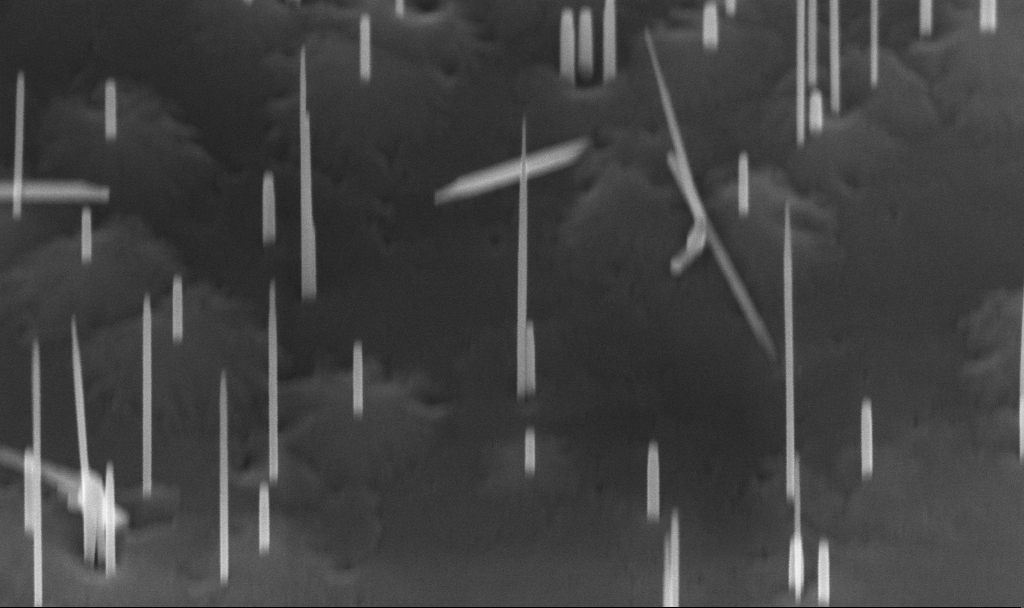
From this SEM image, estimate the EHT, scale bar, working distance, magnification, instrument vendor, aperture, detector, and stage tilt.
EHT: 10 kV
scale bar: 200 nm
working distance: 5.6 mm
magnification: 107.58 K X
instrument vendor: Zeiss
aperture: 30 µm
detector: InLens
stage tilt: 45°